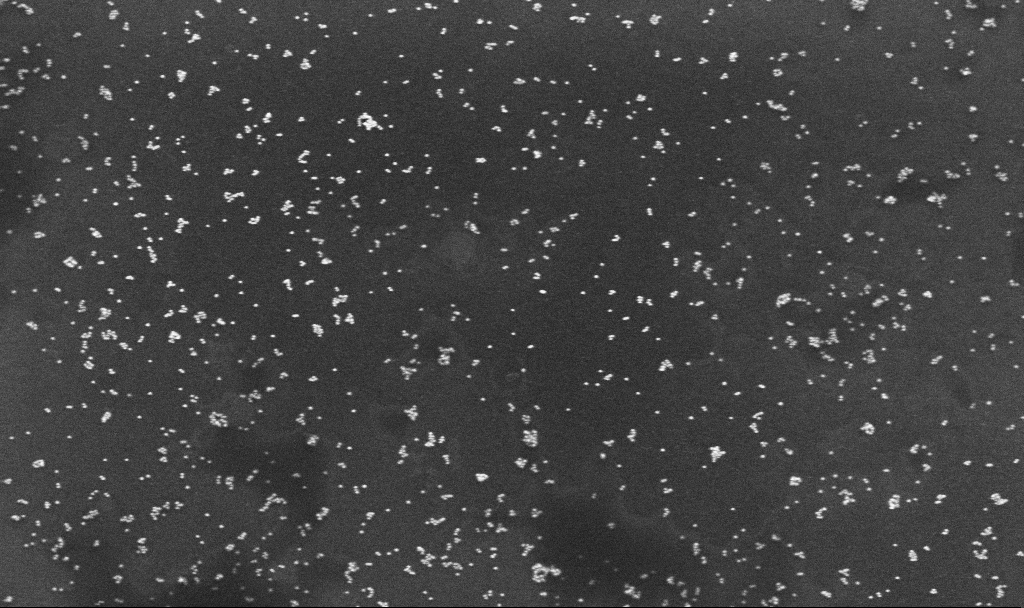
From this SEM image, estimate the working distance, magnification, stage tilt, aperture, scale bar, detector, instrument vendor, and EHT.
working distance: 3.2 mm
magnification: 100 K X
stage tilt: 0°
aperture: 30 µm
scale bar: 200 nm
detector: InLens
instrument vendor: Zeiss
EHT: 10 kV